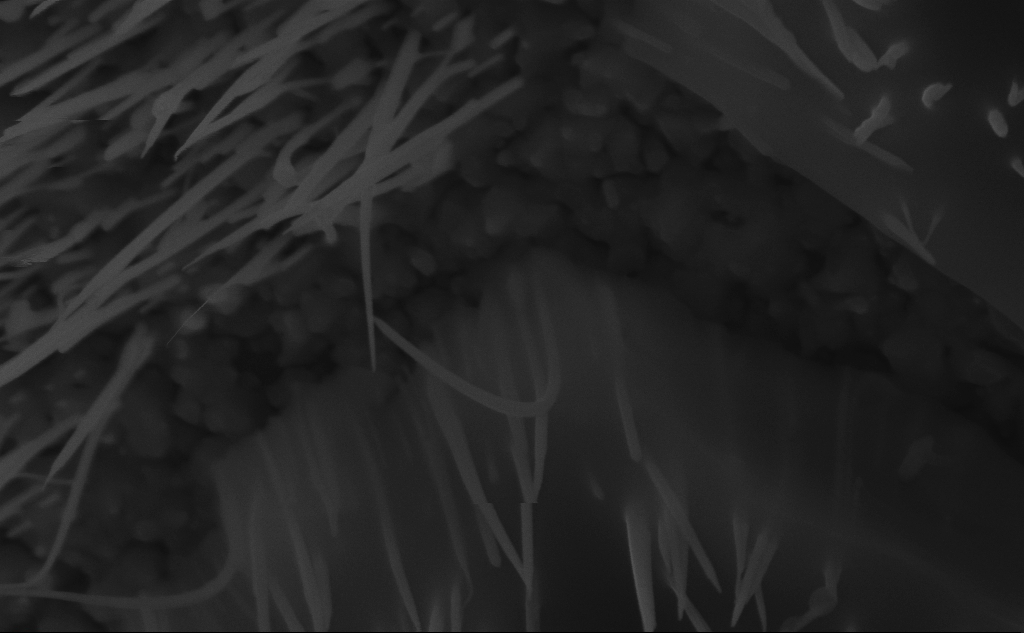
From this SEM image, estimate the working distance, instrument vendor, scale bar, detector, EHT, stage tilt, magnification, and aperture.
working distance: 6 mm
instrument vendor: Zeiss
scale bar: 200 nm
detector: InLens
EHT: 10 kV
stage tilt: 45°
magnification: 98.96 K X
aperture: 30 µm